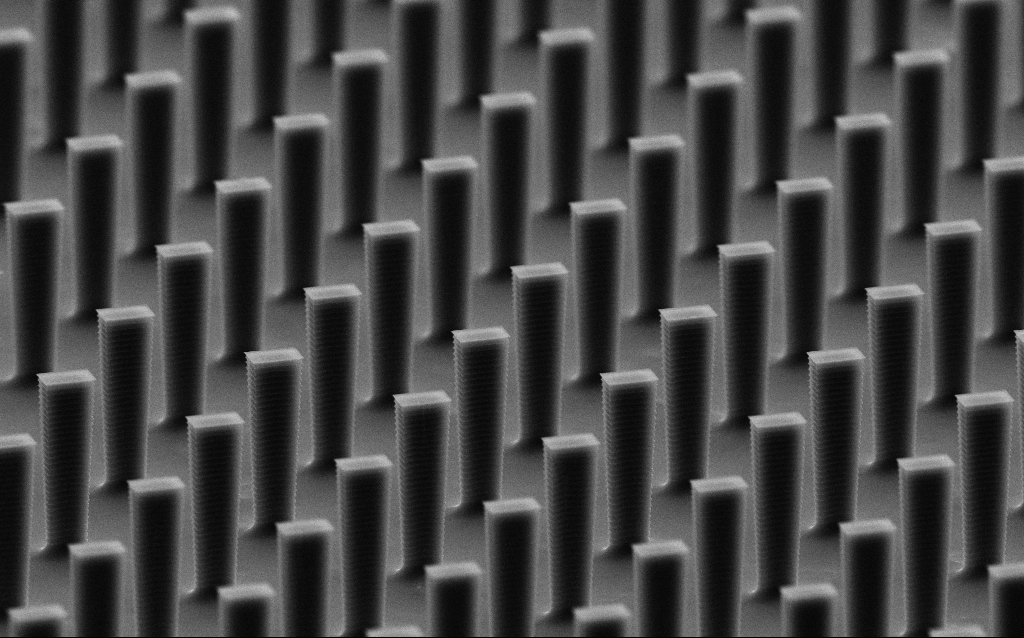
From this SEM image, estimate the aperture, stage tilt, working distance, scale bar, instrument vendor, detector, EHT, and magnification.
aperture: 30 µm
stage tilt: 70°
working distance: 7.5 mm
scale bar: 10000 nm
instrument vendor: Zeiss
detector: SE2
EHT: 10 kV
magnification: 6.6 K X